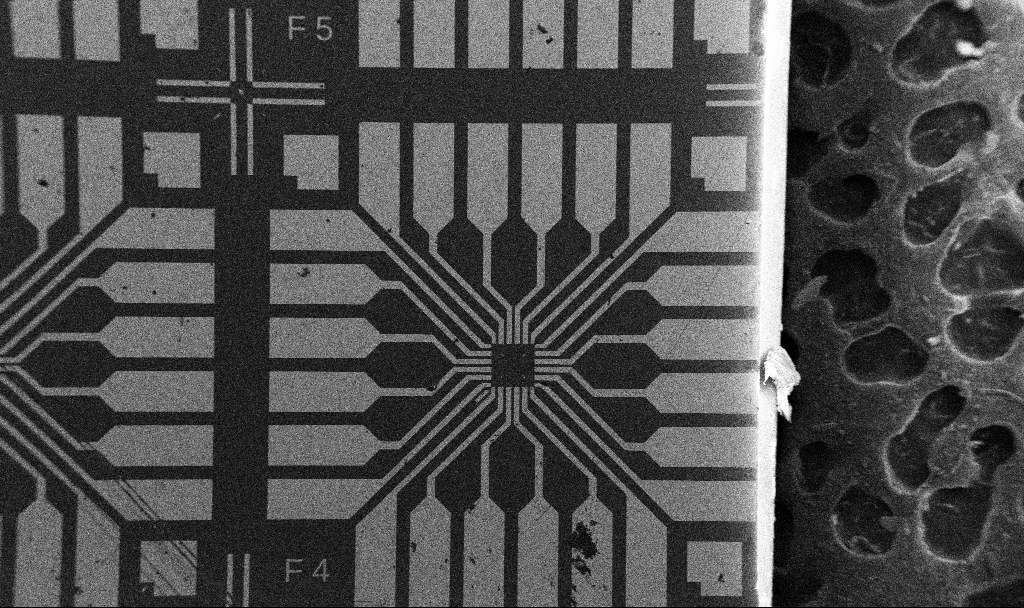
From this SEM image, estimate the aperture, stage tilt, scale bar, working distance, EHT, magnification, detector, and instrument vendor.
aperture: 30 µm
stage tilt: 0°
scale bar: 200000 nm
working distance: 8.9 mm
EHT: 5 kV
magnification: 0.1 K X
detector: SE2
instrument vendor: Zeiss